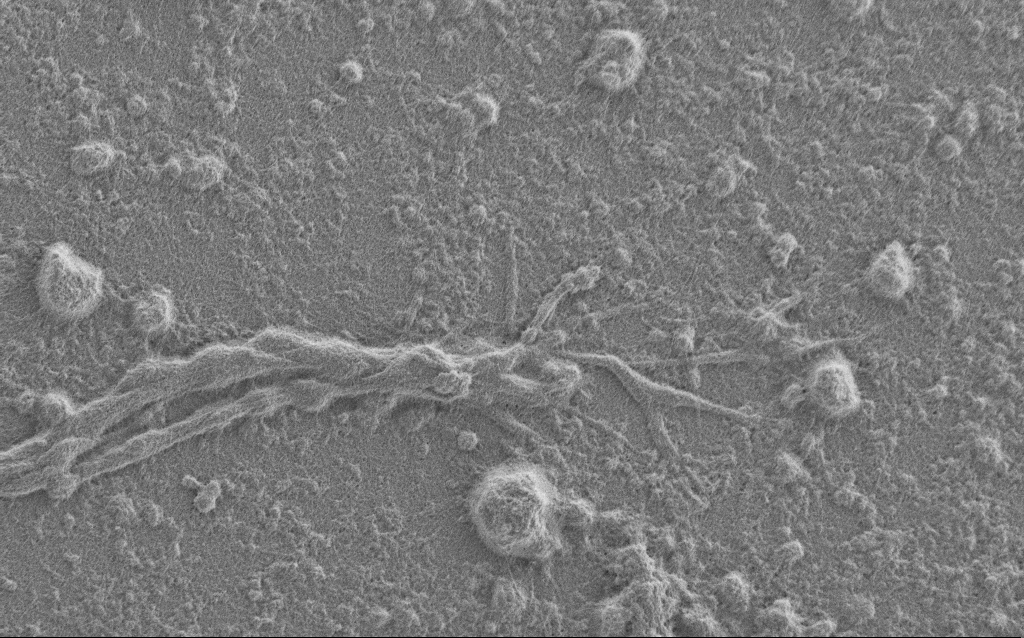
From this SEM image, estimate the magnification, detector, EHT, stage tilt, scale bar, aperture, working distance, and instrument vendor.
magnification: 7.5 K X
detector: SE2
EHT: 1 kV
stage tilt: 0°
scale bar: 2000 nm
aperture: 30 µm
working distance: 6 mm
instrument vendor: Zeiss